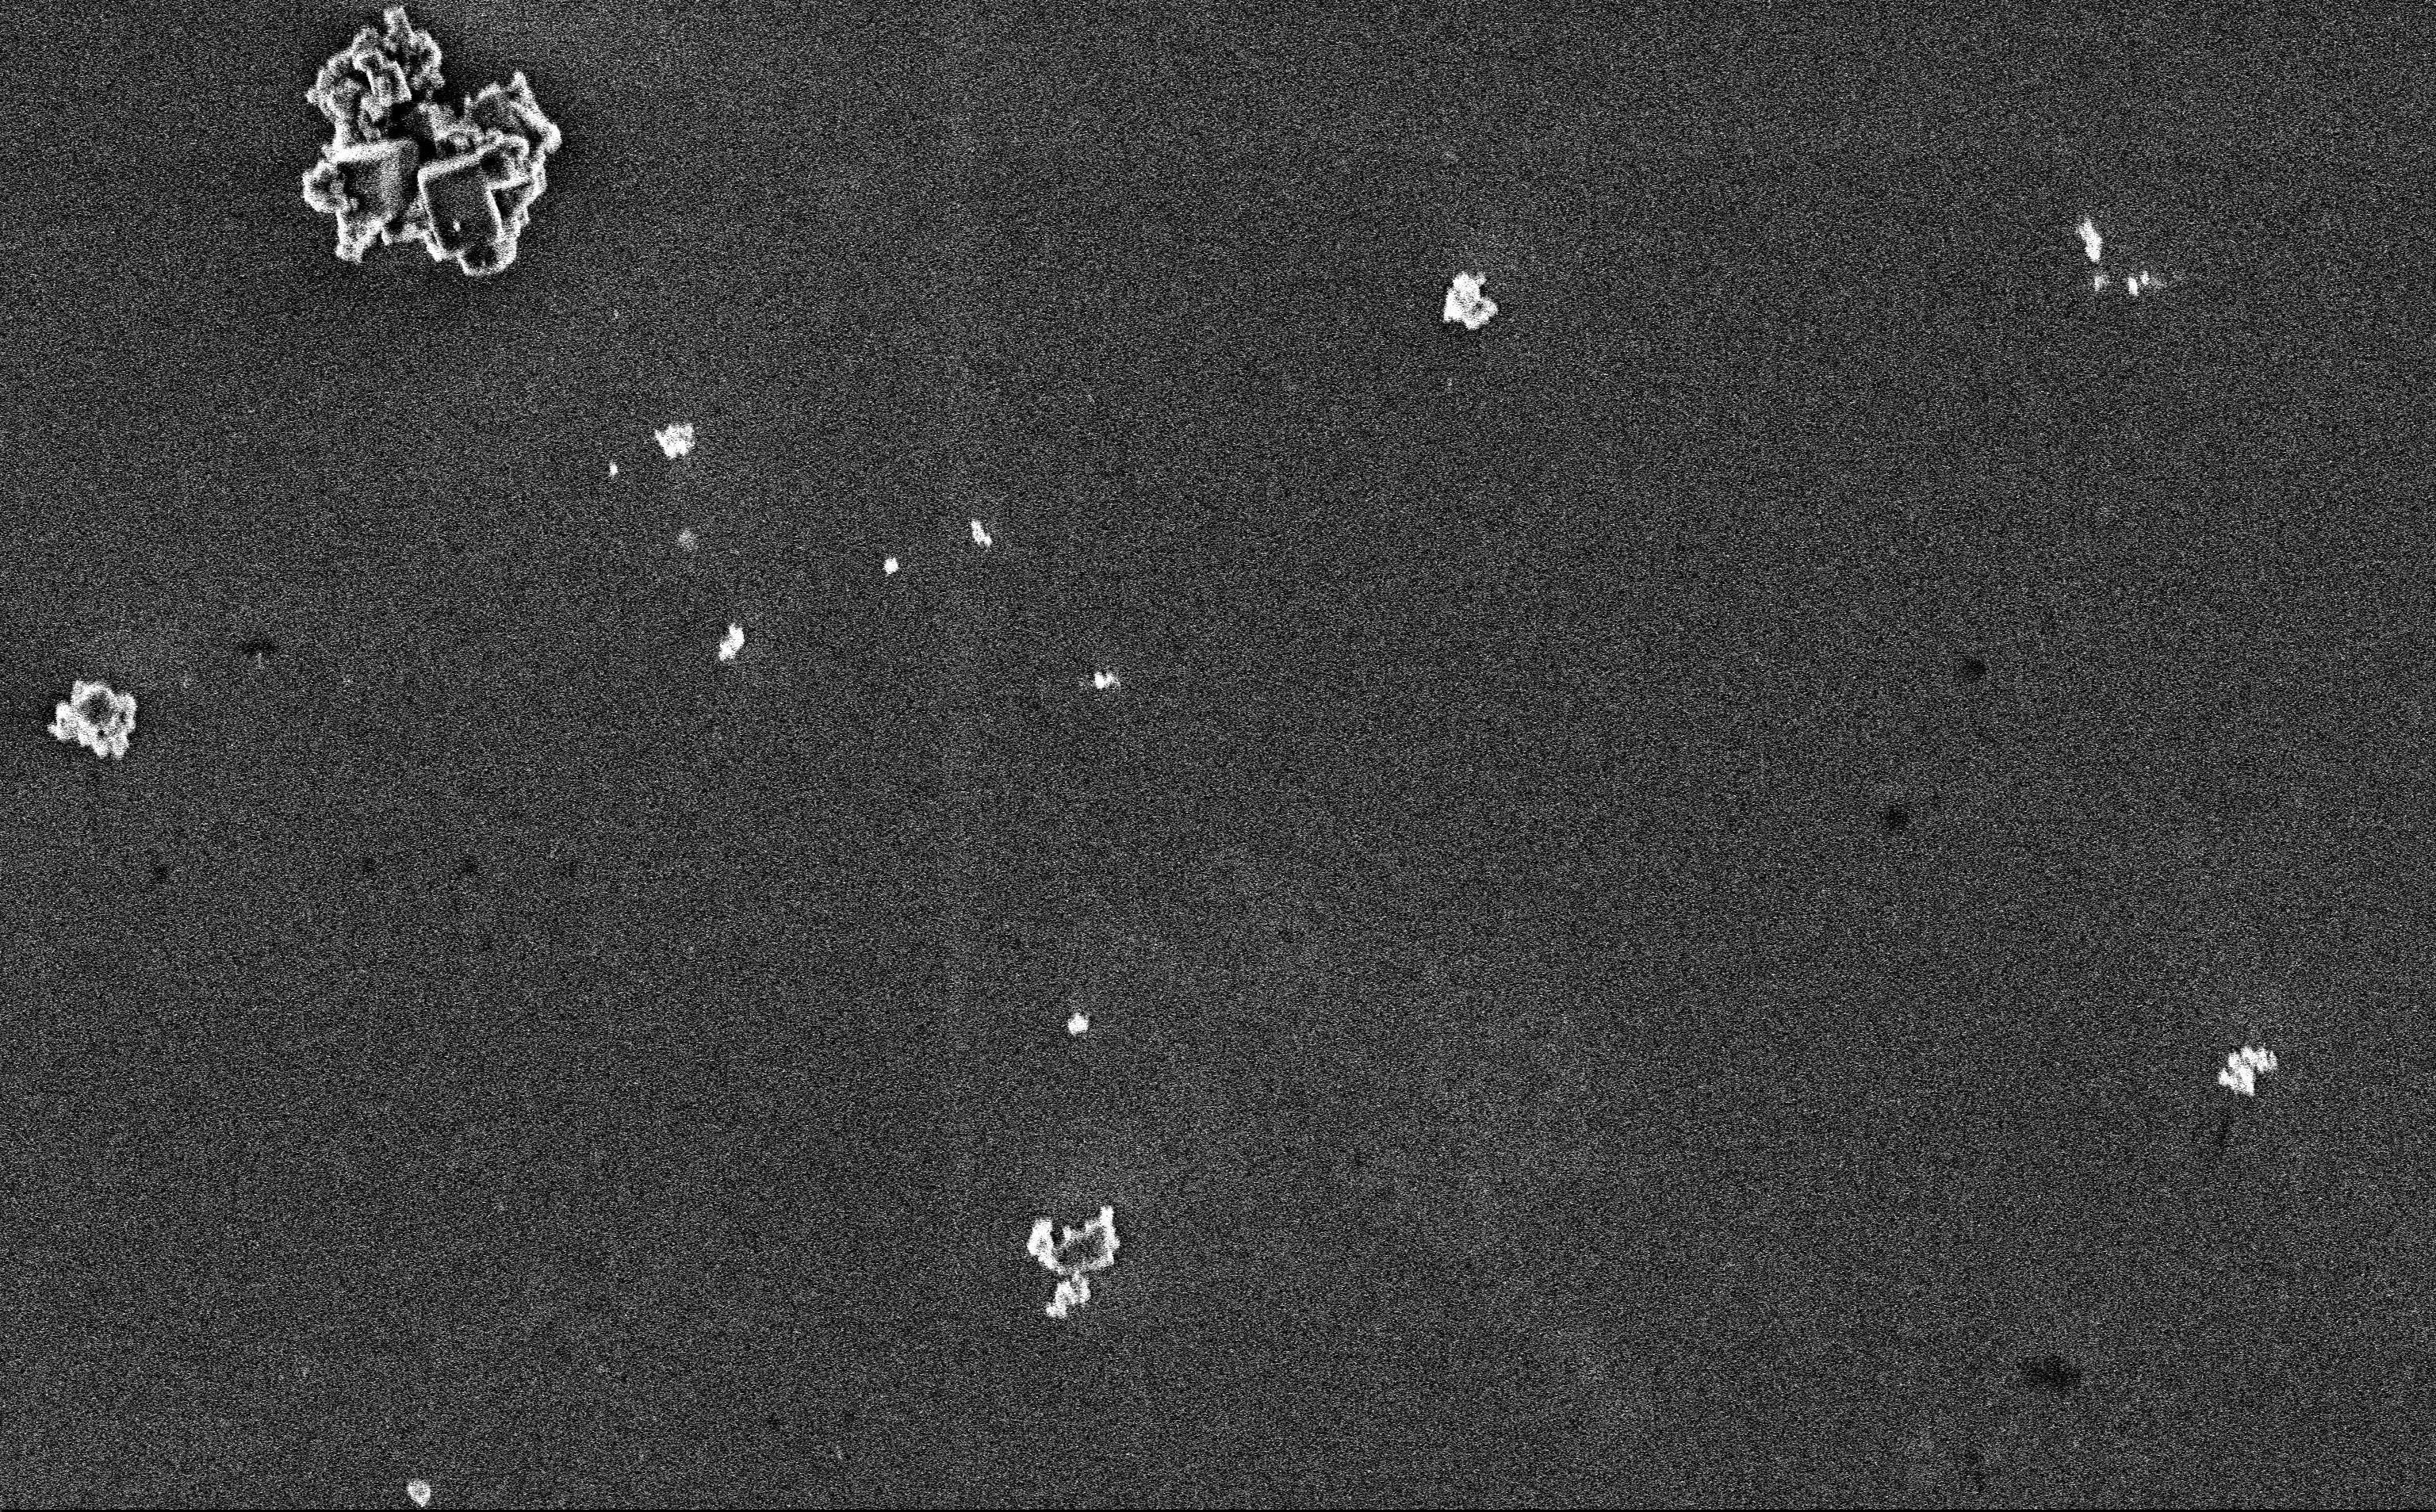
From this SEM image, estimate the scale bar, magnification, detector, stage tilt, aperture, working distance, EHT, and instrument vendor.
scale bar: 2000 nm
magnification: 12.85 K X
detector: InLens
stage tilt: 0°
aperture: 30 µm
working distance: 3 mm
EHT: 3 kV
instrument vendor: Zeiss